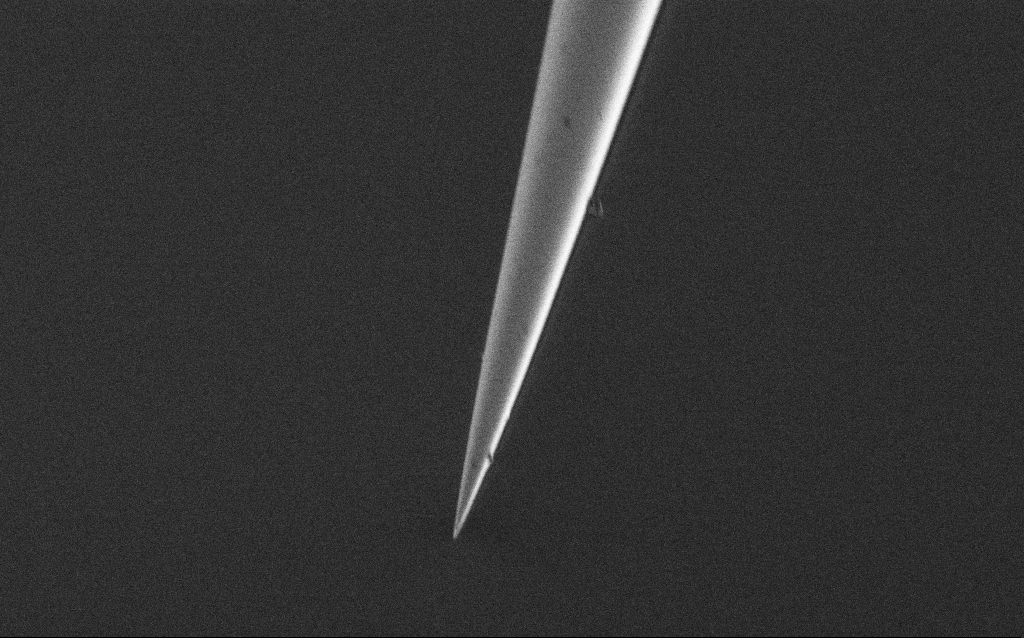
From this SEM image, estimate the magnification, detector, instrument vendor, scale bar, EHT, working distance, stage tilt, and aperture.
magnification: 10 K X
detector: SE2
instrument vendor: Zeiss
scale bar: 2000 nm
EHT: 1 kV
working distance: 6.6 mm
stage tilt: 45°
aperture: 30 µm